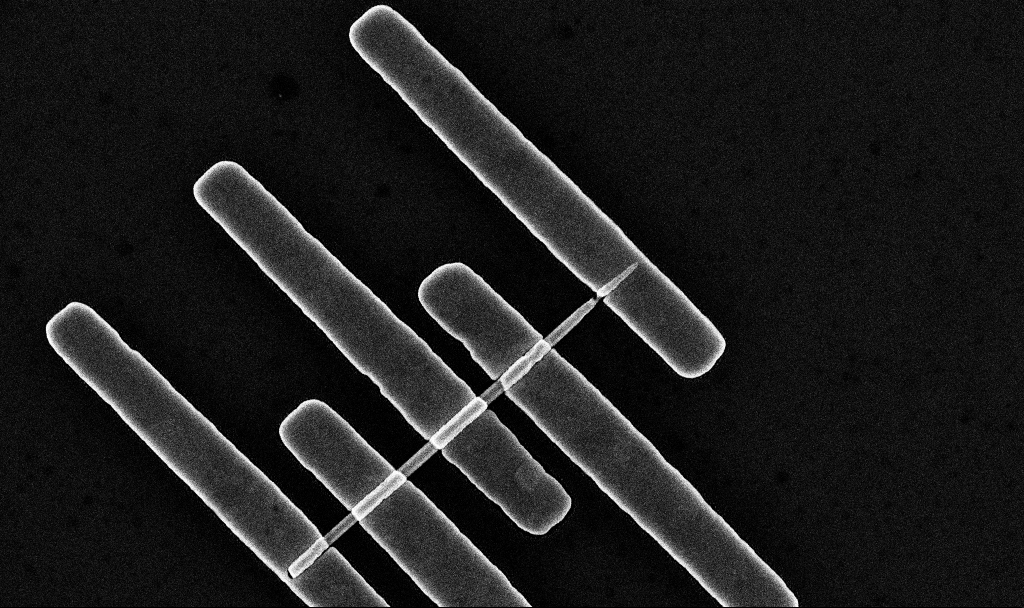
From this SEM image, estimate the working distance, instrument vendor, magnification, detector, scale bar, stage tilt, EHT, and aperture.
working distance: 7.6 mm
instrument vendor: Zeiss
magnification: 34.97 K X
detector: InLens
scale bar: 2000 nm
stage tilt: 0°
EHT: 10 kV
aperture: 30 µm